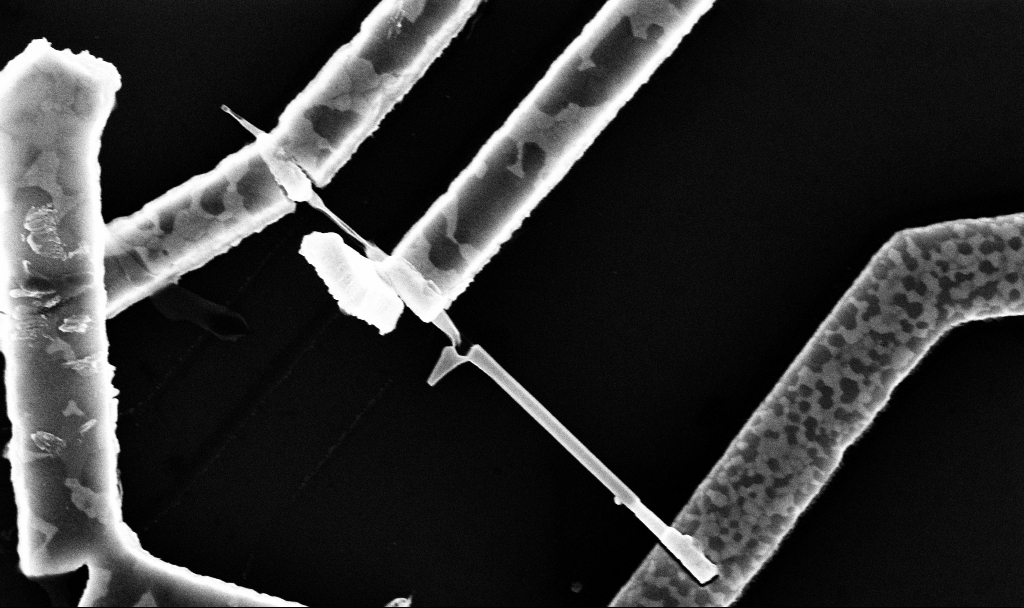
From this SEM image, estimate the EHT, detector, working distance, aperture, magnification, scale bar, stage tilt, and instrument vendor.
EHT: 10 kV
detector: InLens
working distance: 7.7 mm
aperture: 30 µm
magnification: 50.39 K X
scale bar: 1000 nm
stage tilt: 0°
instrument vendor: Zeiss